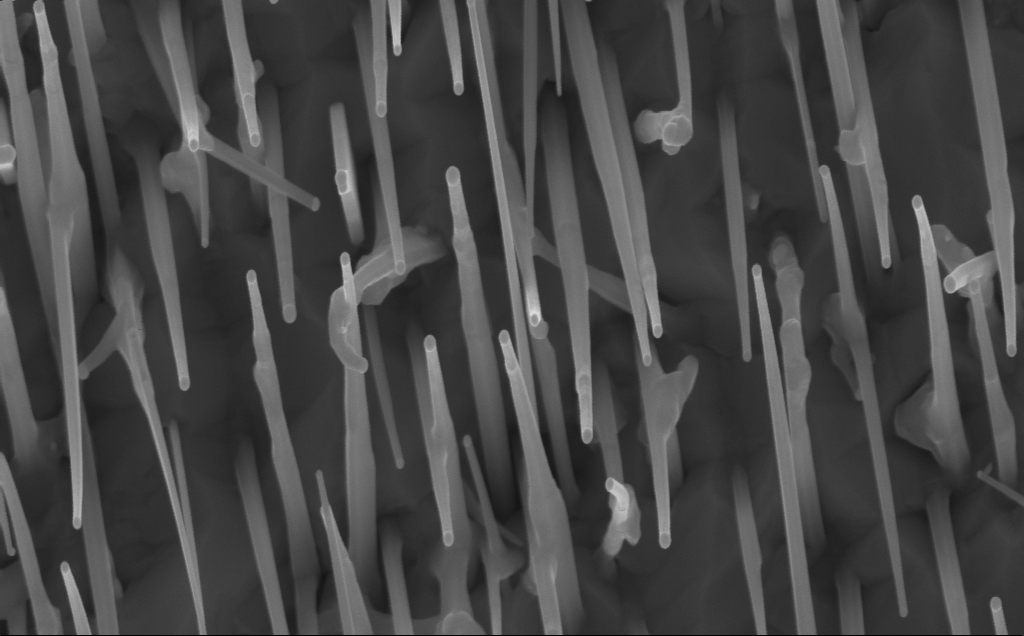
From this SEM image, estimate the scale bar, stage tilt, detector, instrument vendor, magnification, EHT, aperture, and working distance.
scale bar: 200 nm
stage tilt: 0°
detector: InLens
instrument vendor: Zeiss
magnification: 80 K X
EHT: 10 kV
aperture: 30 µm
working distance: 7 mm